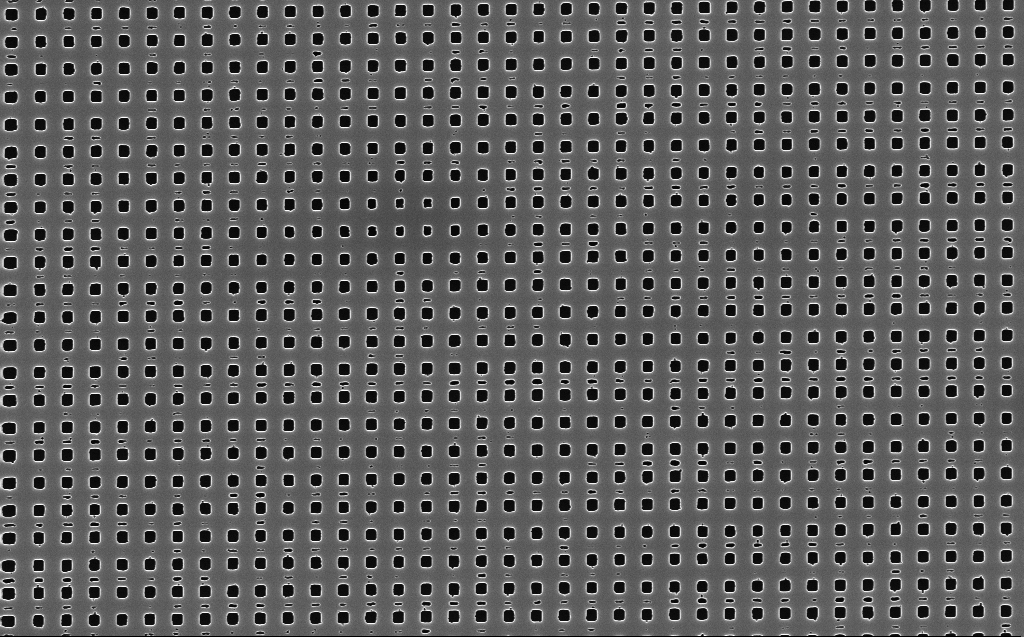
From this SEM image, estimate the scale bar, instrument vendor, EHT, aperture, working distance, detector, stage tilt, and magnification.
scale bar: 1000 nm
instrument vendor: Zeiss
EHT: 10 kV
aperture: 30 µm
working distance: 5 mm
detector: InLens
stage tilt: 0°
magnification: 20.56 K X